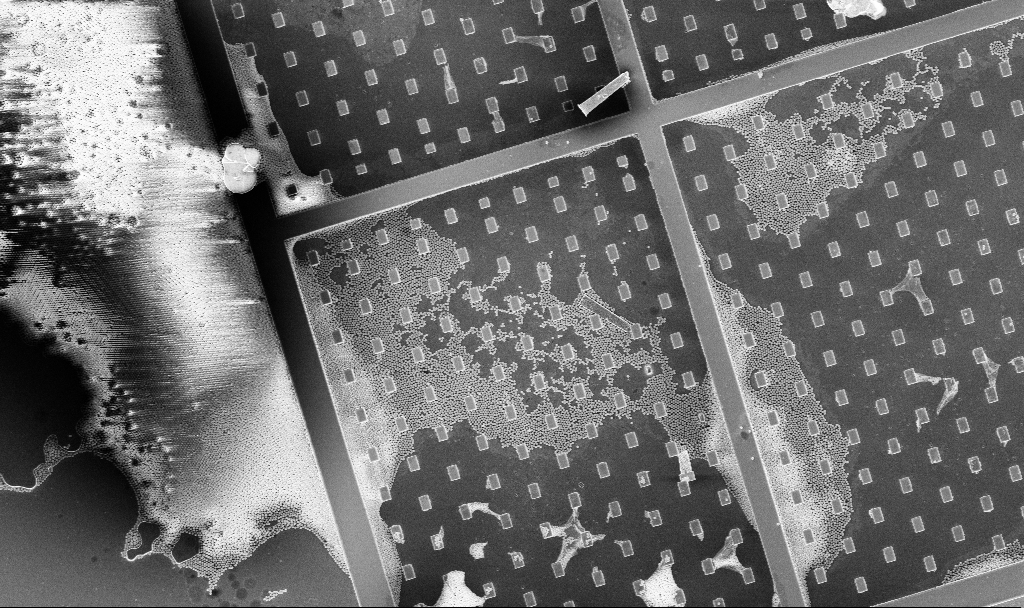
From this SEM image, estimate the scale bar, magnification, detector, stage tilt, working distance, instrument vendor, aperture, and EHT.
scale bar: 20000 nm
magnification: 1.26 K X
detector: InLens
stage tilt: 0°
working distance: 4.9 mm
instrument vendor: Zeiss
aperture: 30 µm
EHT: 5 kV